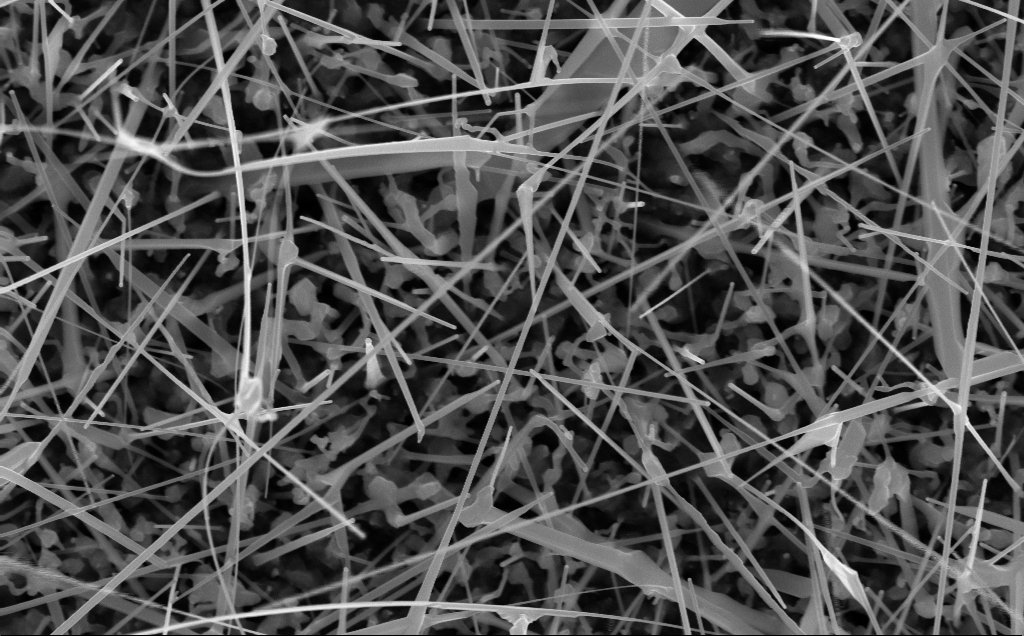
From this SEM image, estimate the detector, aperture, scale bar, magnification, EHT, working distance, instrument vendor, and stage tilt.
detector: InLens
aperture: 30 µm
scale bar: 1000 nm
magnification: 46.59 K X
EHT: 10 kV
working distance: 8 mm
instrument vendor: Zeiss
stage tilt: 0°